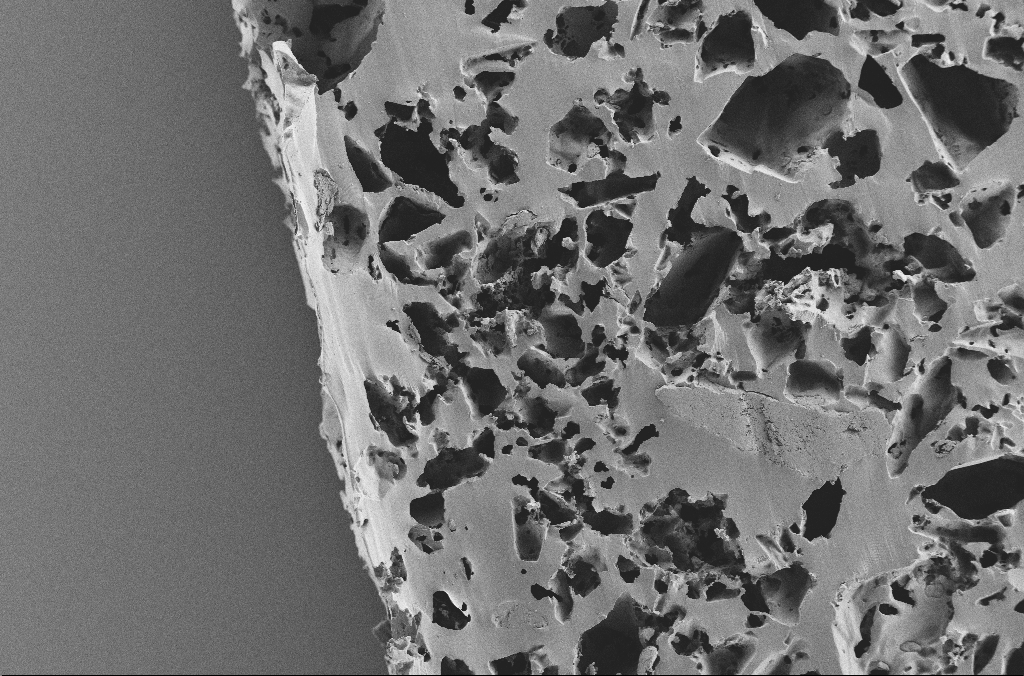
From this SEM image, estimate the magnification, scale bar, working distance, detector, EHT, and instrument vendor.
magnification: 0.25 K X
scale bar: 100000 nm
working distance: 3.1 mm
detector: SE2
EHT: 2 kV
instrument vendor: Zeiss